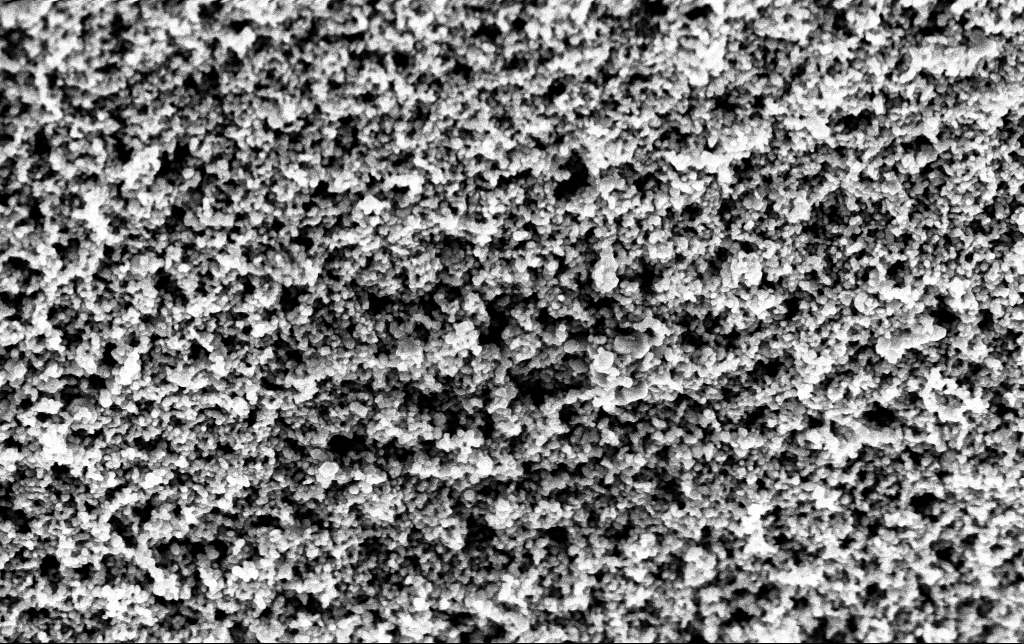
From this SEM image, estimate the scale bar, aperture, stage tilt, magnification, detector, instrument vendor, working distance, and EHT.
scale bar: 200 nm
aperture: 30 µm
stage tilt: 0°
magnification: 80 K X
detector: InLens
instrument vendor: Zeiss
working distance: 2.8 mm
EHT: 3 kV